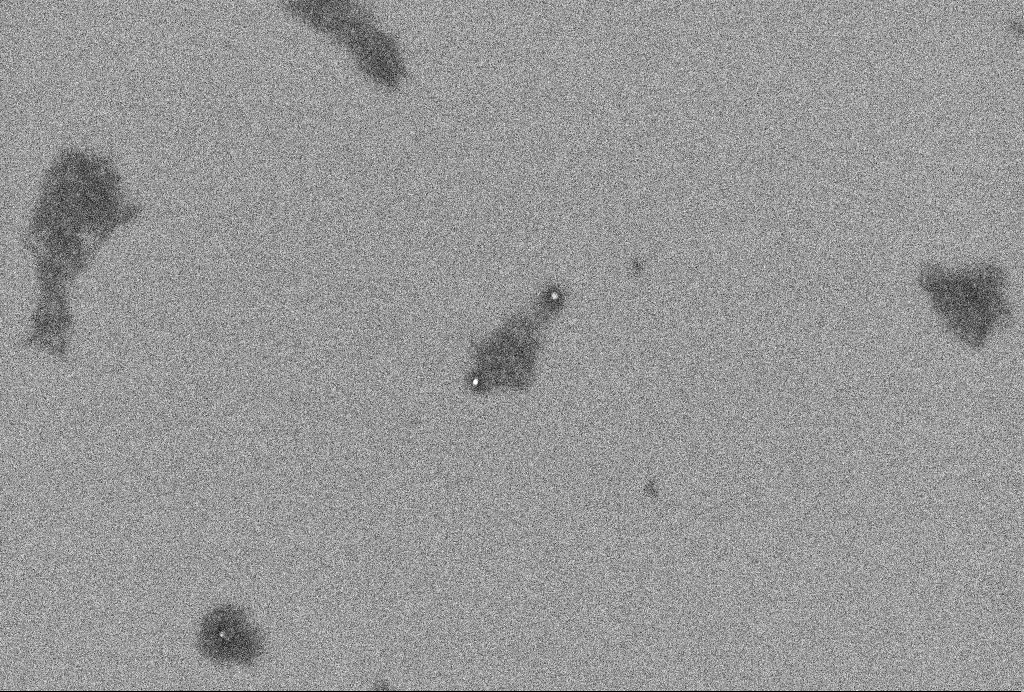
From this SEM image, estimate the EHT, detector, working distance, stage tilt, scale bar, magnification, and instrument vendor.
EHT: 2 kV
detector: InLens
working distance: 3.3 mm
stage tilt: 0°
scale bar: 200 nm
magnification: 64.42 K X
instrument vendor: Zeiss